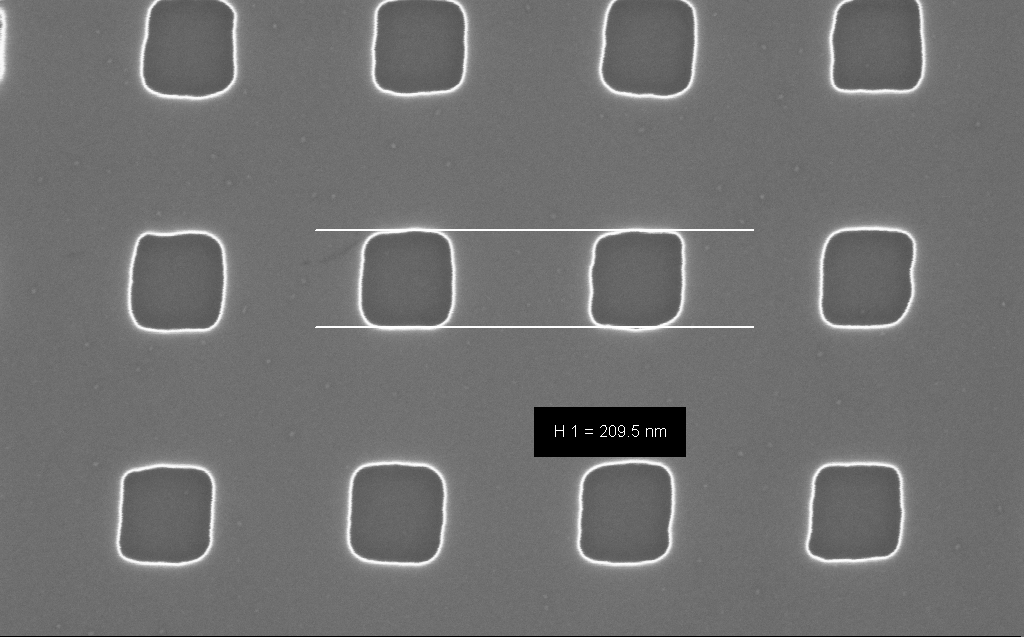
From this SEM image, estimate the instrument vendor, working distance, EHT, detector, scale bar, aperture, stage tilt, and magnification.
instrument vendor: Zeiss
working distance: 7 mm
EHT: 10 kV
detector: InLens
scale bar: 200 nm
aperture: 30 µm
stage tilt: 0°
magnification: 170 K X